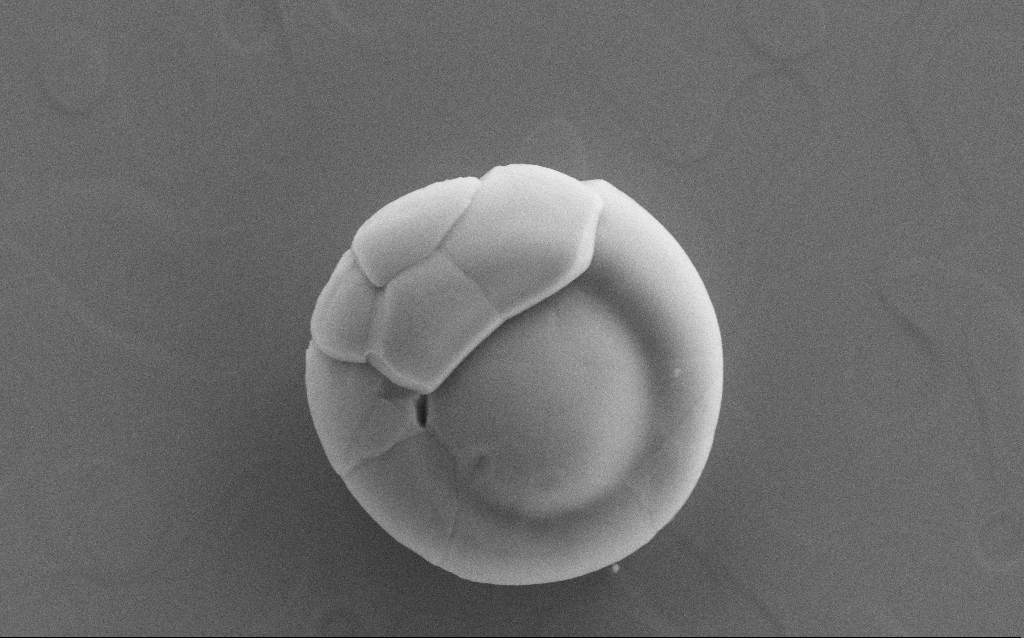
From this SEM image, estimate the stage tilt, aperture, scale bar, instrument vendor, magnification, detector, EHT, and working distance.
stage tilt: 0°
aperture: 30 µm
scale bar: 1000 nm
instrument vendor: Zeiss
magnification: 37.12 K X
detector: SE2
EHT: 10 kV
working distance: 2 mm